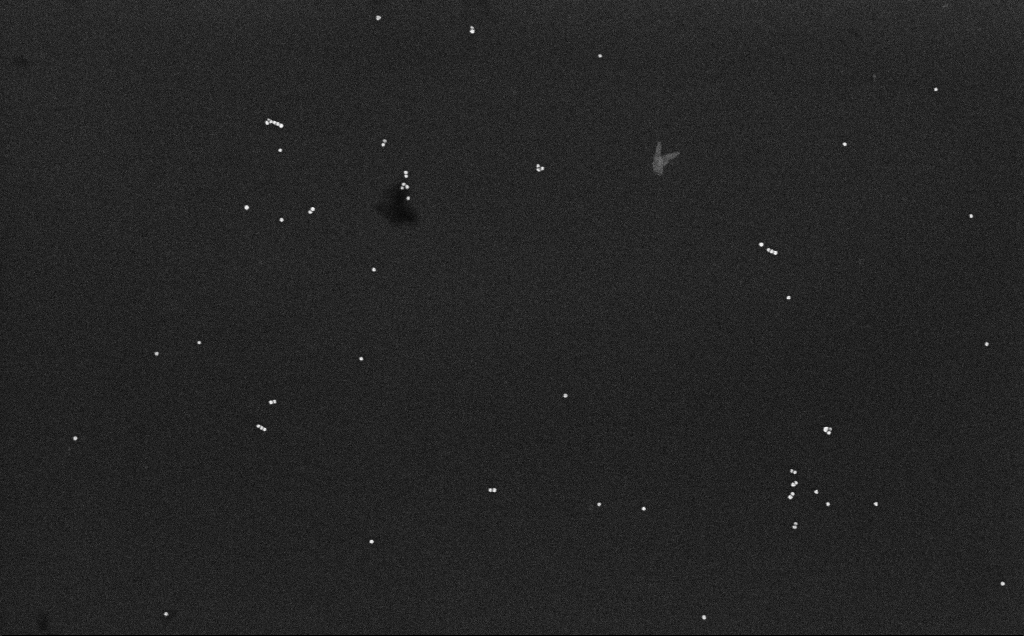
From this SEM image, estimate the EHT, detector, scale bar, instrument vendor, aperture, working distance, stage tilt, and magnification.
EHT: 10 kV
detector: InLens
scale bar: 200 nm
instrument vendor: Zeiss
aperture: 30 µm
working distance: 6.6 mm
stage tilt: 0°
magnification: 100 K X